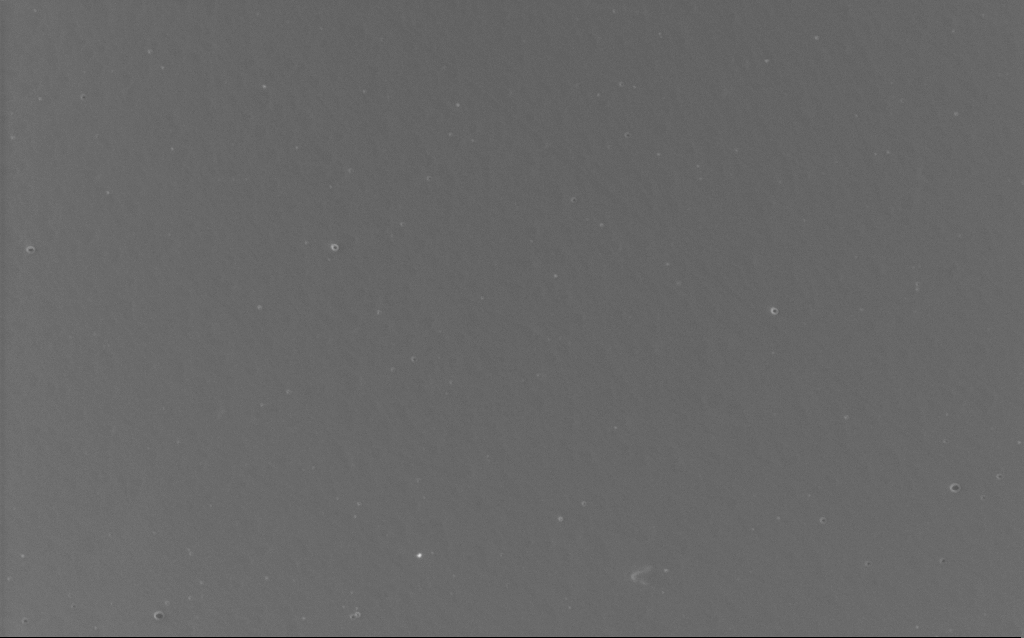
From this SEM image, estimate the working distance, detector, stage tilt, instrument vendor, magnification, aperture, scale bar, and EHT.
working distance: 2 mm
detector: InLens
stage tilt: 0°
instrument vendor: Zeiss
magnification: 1.32 K X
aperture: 30 µm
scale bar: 20000 nm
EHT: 10 kV